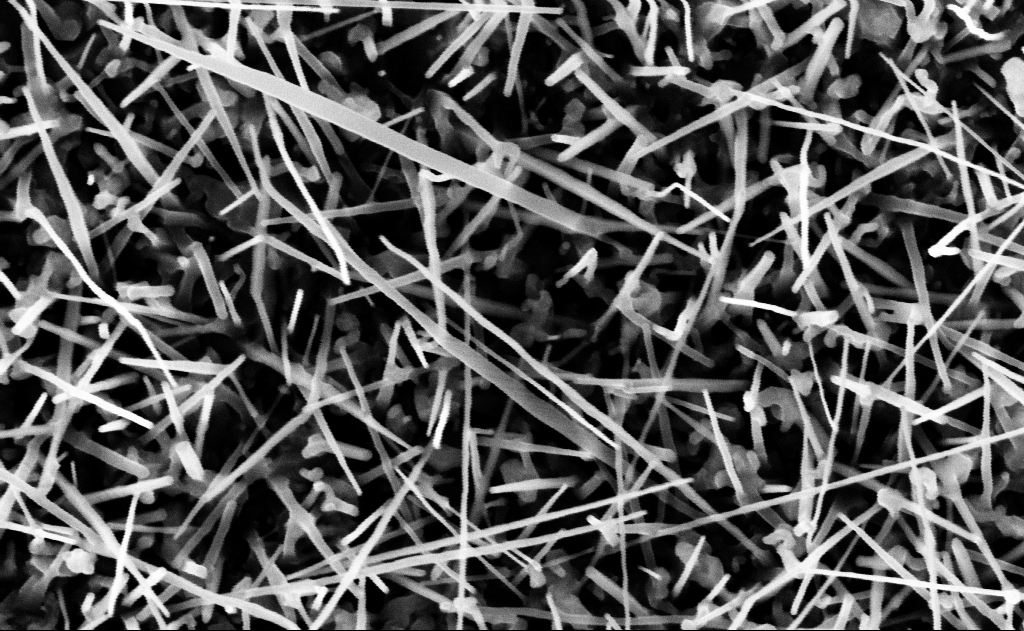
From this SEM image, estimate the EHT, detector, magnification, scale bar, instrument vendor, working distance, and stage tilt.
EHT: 10 kV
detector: InLens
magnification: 80 K X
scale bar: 200 nm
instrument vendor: Zeiss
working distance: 15 mm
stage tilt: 0°